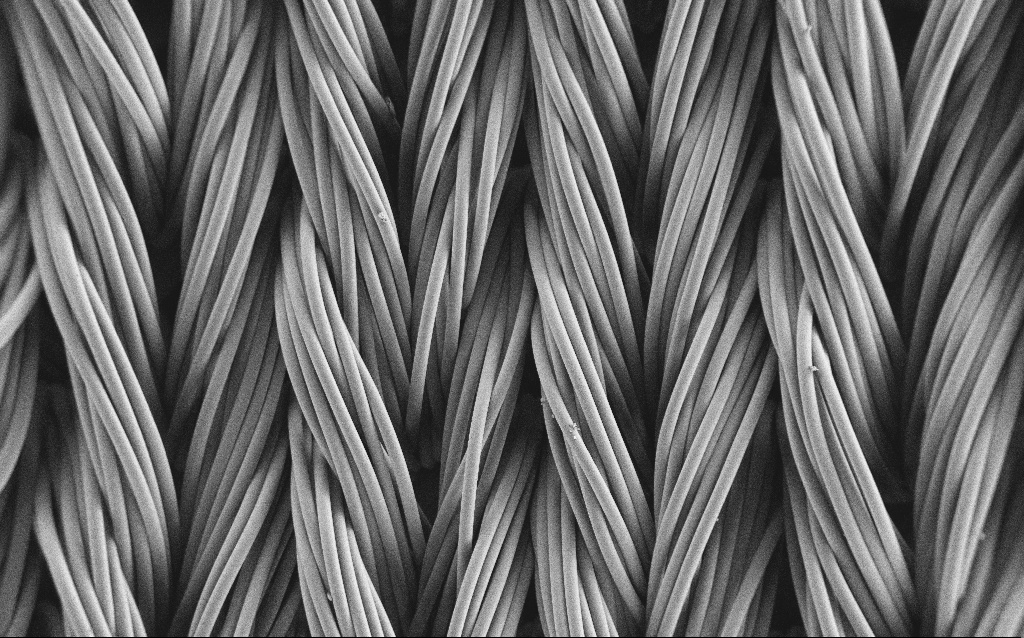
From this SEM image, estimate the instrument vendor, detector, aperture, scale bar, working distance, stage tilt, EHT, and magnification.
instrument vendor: Zeiss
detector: SE2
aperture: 30 µm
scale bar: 100000 nm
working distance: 4 mm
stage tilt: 0°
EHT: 1 kV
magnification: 0.209 K X